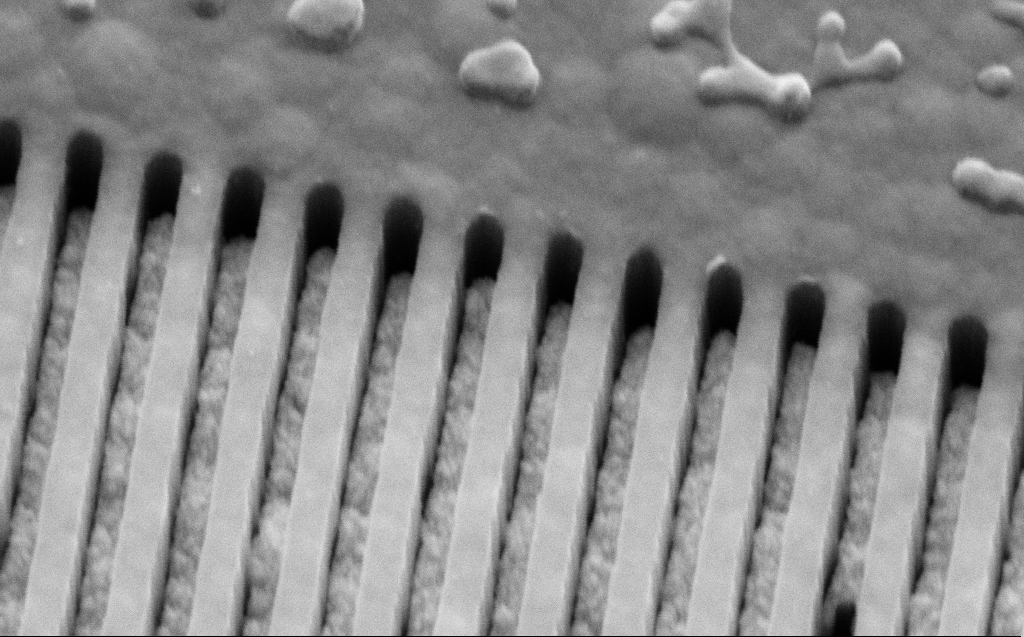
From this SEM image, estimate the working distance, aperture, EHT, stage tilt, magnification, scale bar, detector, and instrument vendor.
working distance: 9 mm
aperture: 30 µm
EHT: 5 kV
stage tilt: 45°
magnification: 131.5 K X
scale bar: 200 nm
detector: SE2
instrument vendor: Zeiss